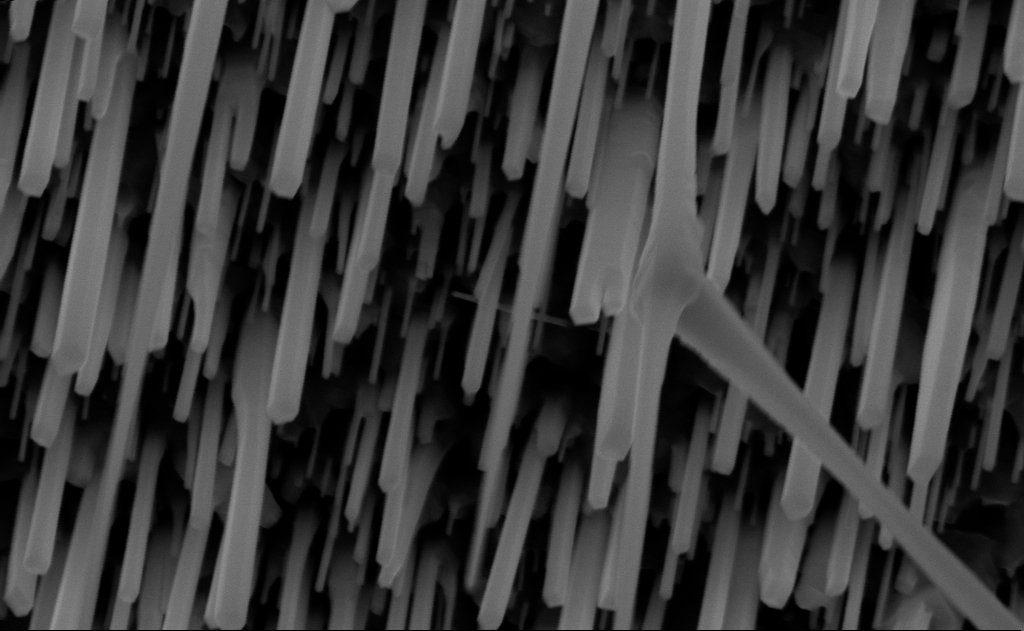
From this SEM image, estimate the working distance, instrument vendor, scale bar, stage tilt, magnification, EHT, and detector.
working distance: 9 mm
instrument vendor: Zeiss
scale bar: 200 nm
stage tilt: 0°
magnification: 80 K X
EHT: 10 kV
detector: InLens